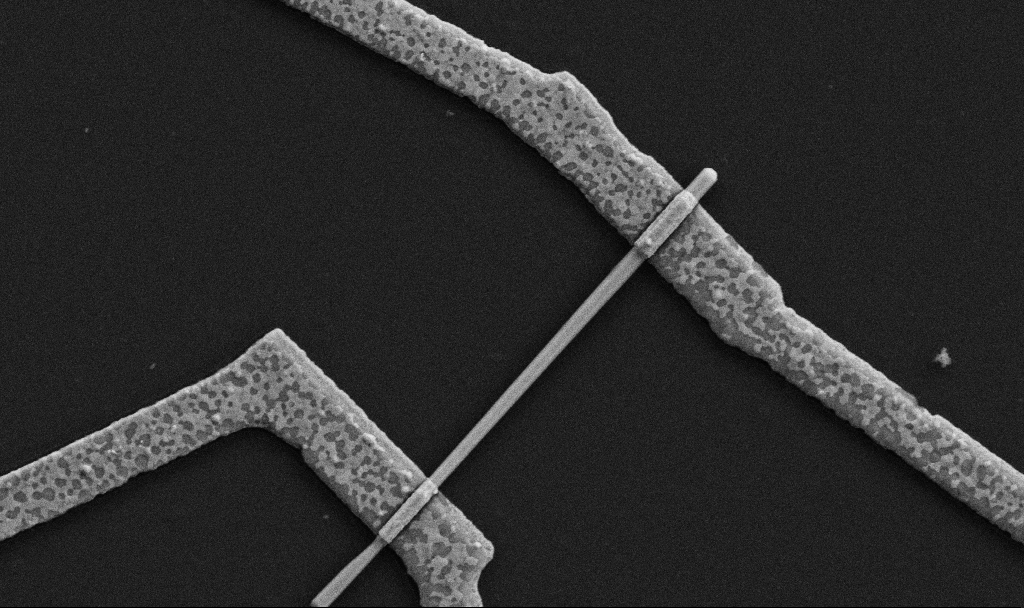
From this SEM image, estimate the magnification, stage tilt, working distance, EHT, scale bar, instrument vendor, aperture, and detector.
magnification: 30 K X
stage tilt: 0°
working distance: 8.7 mm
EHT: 5 kV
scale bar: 1000 nm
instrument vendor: Zeiss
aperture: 30 µm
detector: SE2